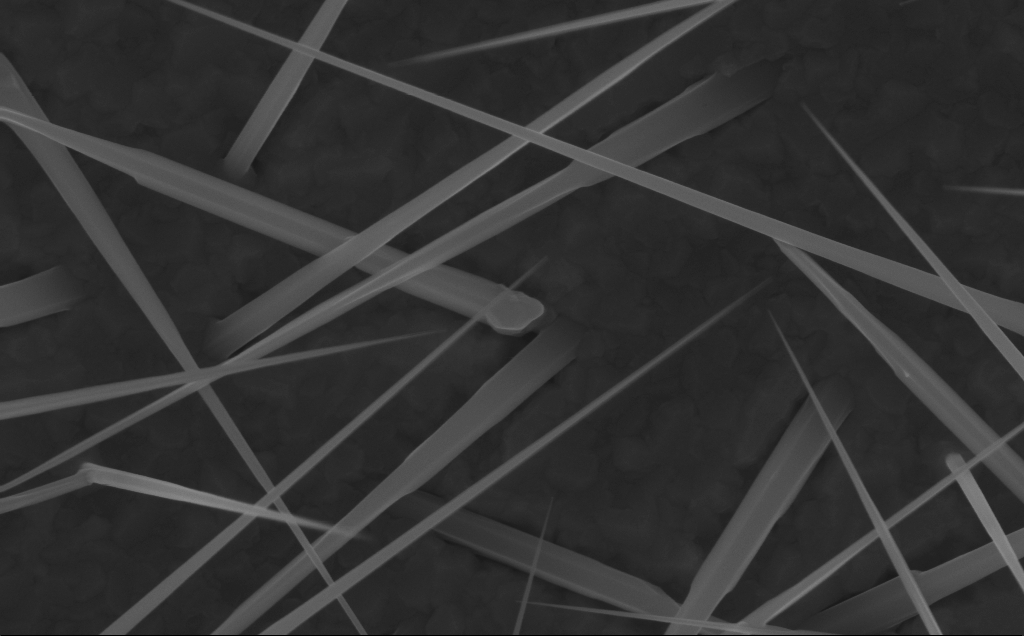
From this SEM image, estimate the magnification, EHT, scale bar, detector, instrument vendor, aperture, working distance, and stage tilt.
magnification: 80 K X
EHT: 10 kV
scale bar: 200 nm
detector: InLens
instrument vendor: Zeiss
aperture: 30 µm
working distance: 6 mm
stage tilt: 0°